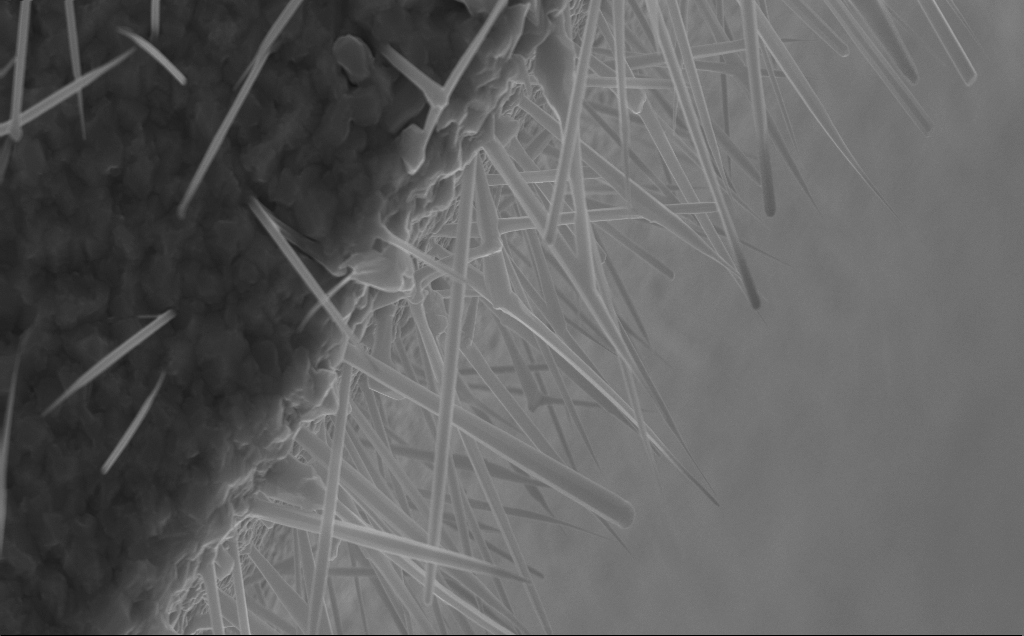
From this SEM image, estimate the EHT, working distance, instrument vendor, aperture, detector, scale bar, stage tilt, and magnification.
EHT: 10 kV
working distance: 5 mm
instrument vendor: Zeiss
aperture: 30 µm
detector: InLens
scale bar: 2000 nm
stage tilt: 0°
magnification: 32.35 K X